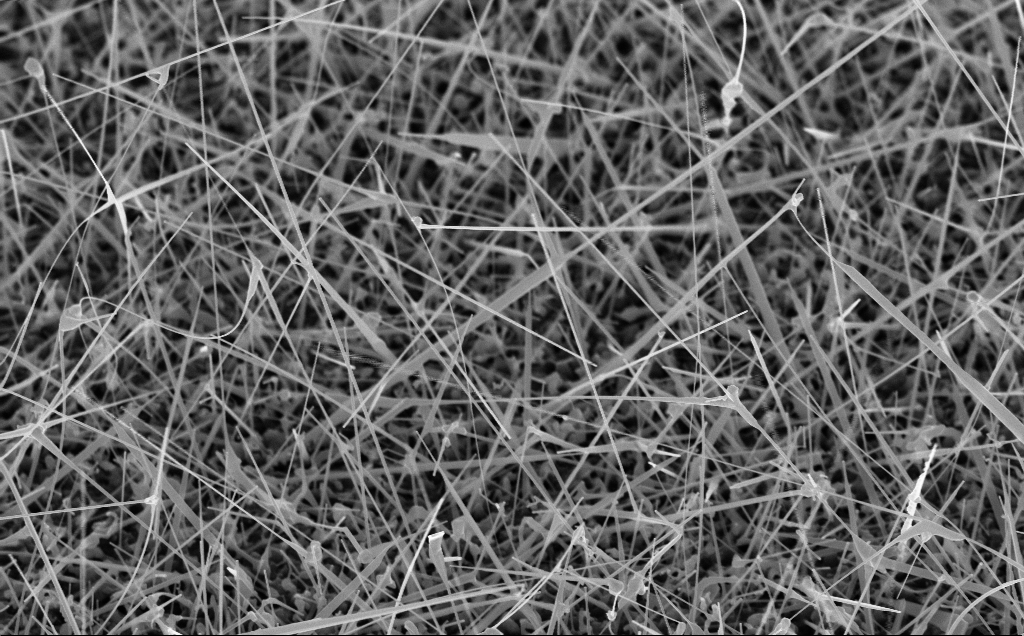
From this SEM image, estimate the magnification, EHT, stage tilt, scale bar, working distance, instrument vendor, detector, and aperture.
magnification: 25.4 K X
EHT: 10 kV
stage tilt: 45°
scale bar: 2000 nm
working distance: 8 mm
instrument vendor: Zeiss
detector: InLens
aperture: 30 µm